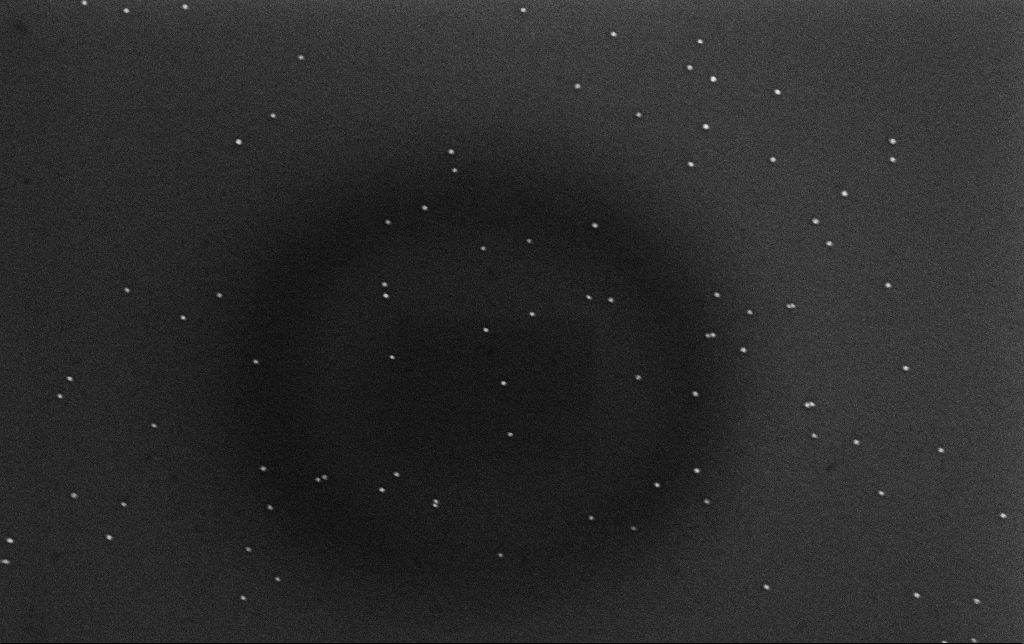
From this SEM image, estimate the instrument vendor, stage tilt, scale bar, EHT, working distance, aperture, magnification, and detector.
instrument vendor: Zeiss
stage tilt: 0°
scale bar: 200 nm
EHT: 10 kV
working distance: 3.2 mm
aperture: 30 µm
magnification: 100 K X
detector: InLens